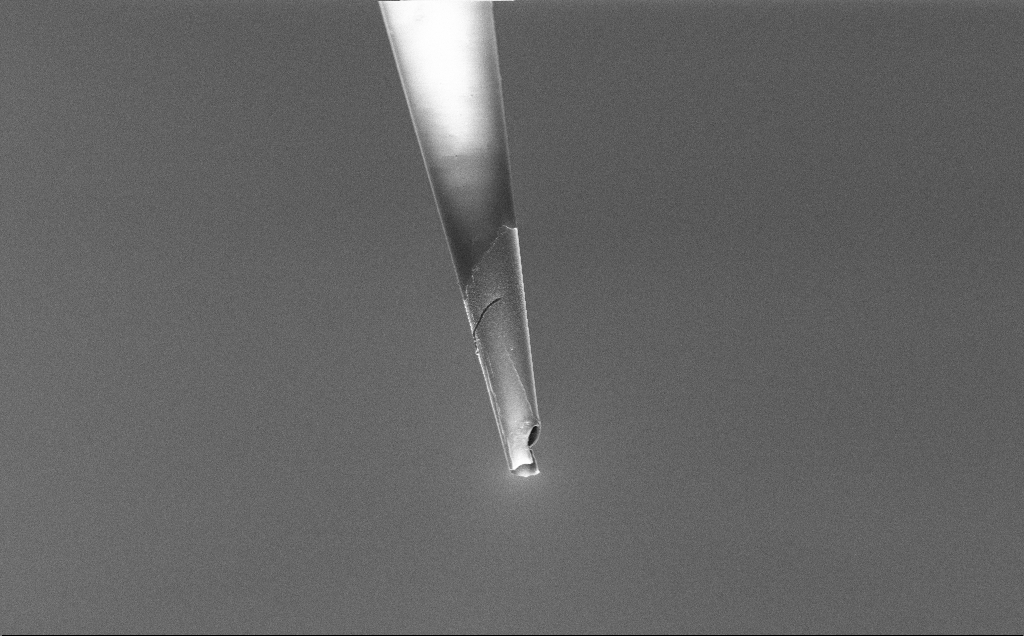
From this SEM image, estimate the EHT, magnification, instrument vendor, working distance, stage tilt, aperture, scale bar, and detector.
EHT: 3 kV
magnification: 3 K X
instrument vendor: Zeiss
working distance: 7.8 mm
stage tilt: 45°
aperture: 30 µm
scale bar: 10000 nm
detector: InLens